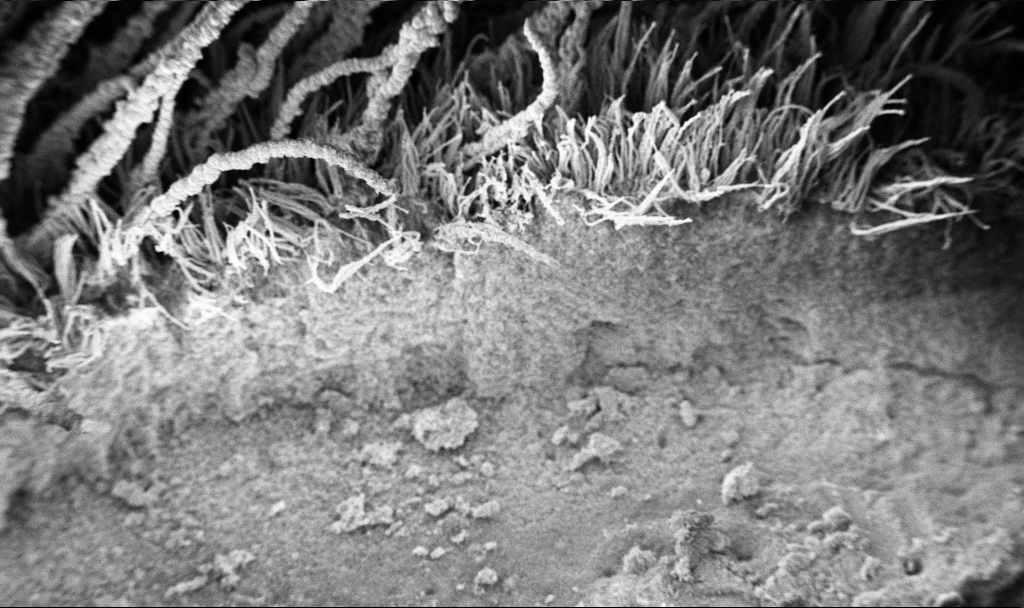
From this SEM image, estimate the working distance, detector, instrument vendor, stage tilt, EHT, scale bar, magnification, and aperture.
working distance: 7.5 mm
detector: InLens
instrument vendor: Zeiss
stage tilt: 37.7°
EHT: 3 kV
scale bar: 100000 nm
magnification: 0.15 K X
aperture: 30 µm